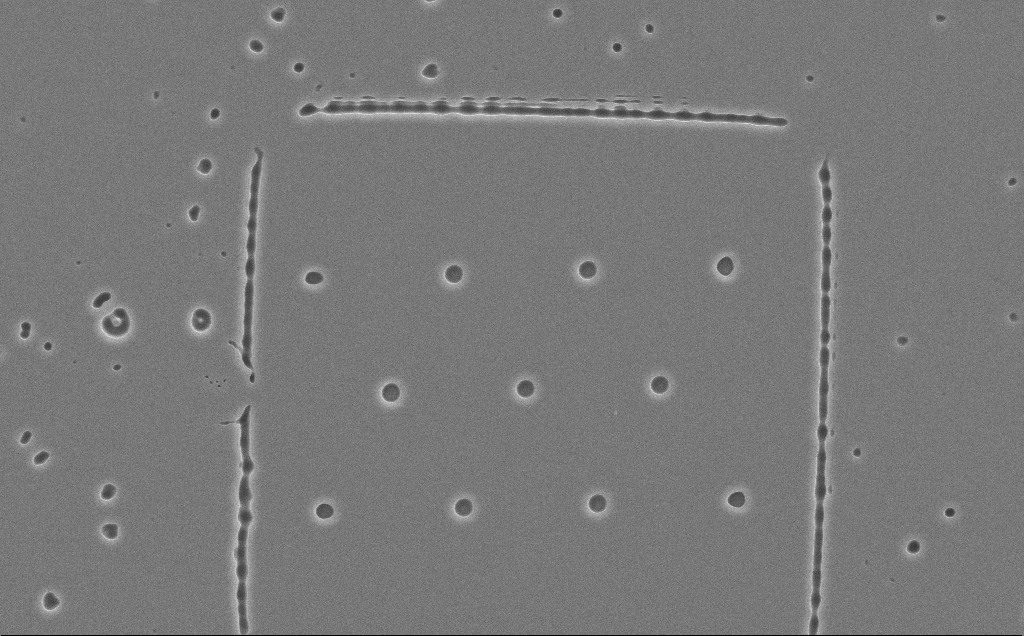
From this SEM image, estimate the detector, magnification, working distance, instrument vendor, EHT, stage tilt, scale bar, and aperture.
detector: SE2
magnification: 2.44 K X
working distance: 13 mm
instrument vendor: Zeiss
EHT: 10 kV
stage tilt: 0°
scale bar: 10000 nm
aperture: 30 µm